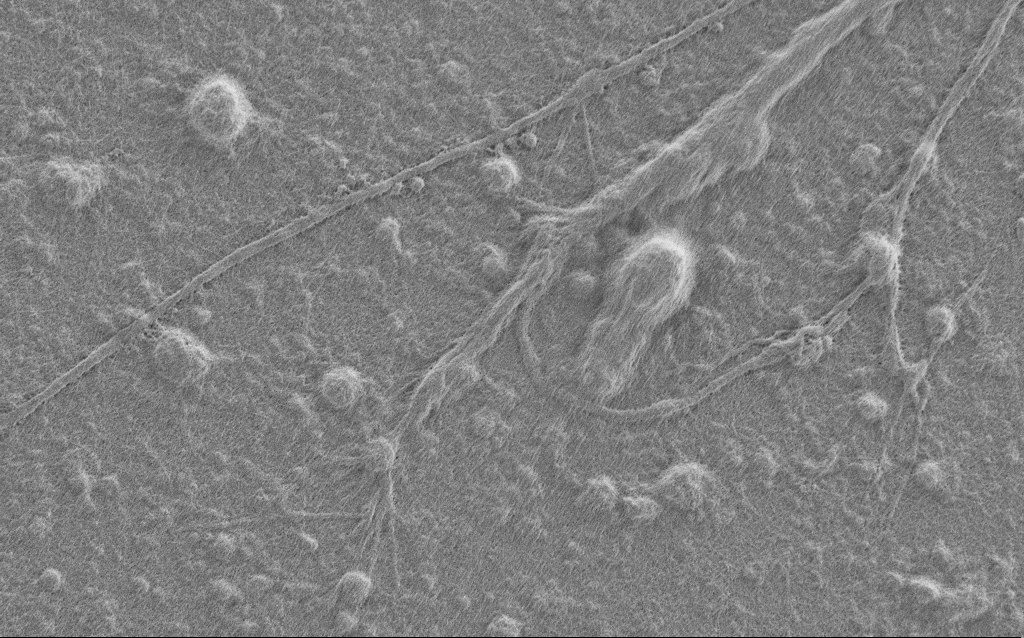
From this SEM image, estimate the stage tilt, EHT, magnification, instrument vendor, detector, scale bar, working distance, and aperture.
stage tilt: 0°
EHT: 1 kV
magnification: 7.5 K X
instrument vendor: Zeiss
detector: SE2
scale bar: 2000 nm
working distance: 6 mm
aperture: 30 µm